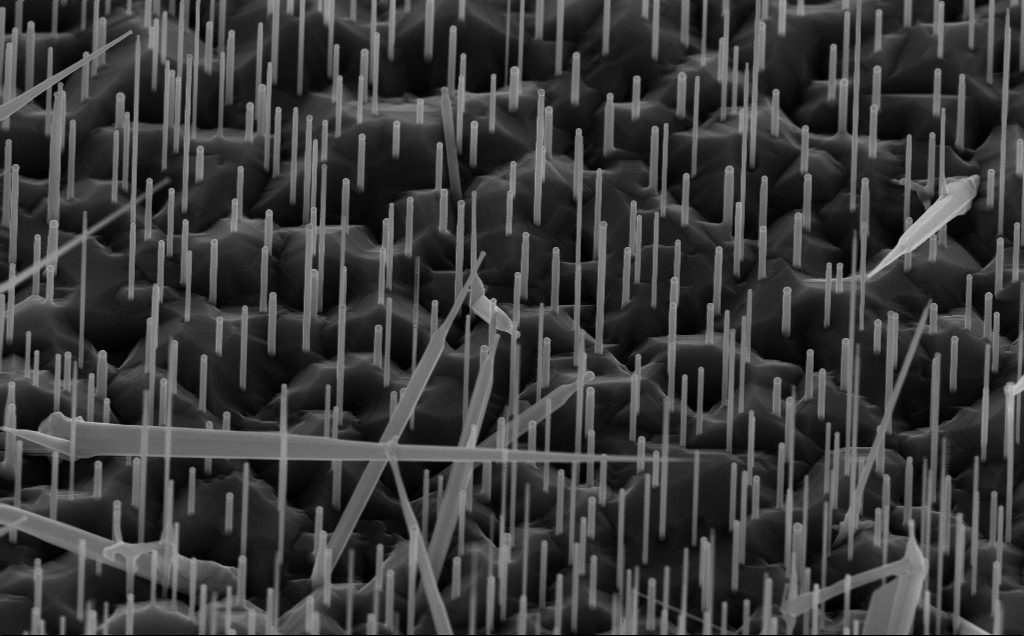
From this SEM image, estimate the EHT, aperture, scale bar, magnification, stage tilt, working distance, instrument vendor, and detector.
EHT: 10 kV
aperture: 30 µm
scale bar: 1000 nm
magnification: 40 K X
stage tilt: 45°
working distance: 7 mm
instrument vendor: Zeiss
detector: InLens